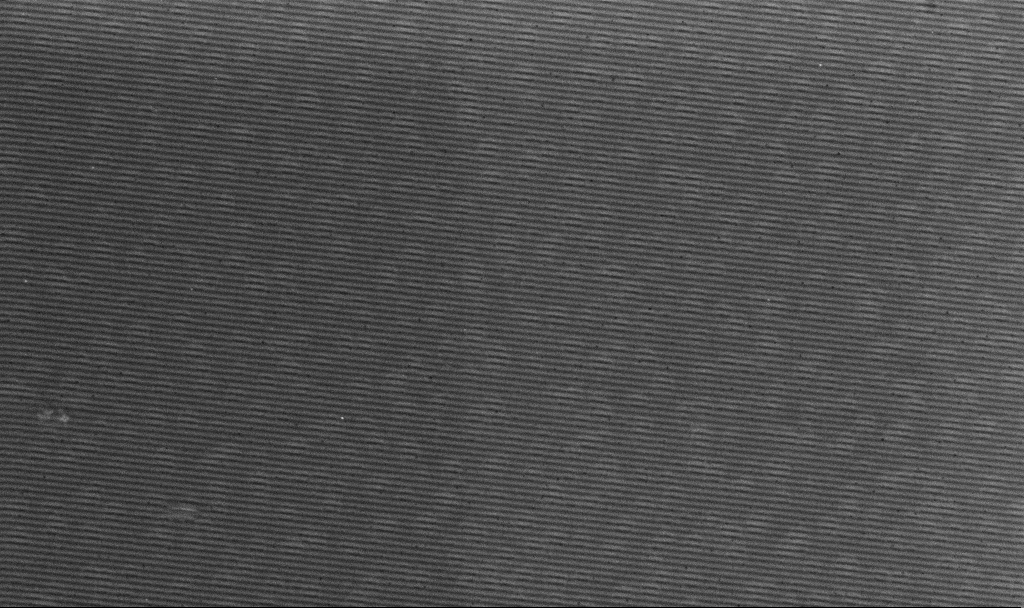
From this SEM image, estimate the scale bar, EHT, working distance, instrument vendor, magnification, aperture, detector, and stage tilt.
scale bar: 2000 nm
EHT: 3 kV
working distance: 2.9 mm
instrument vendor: Zeiss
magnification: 10 K X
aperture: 30 µm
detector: InLens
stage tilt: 0°